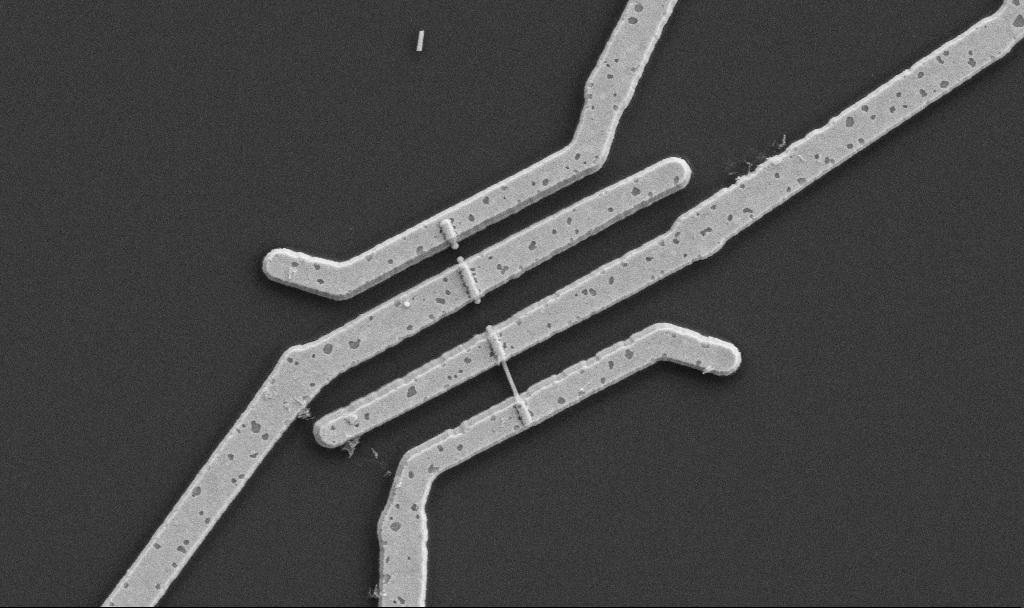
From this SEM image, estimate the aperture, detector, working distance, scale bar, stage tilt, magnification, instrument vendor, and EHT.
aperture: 30 µm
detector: SE2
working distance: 10.7 mm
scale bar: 2000 nm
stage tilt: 0°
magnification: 20 K X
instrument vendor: Zeiss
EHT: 5 kV